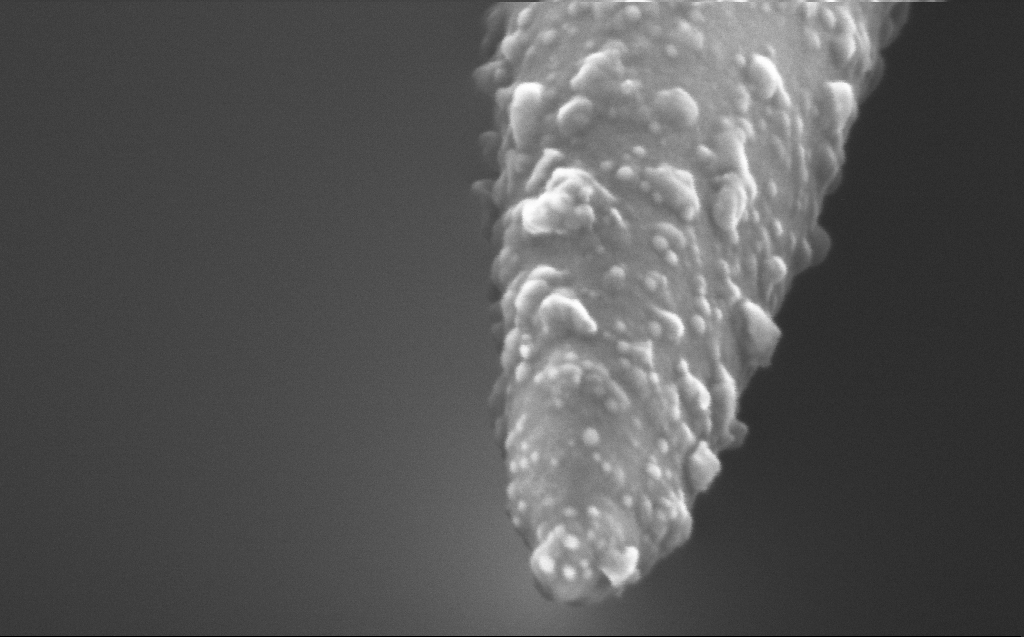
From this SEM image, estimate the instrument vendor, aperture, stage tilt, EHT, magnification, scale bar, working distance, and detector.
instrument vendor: Zeiss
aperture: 30 µm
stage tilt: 45°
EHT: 5 kV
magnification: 400 K X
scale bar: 100 nm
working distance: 3 mm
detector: InLens